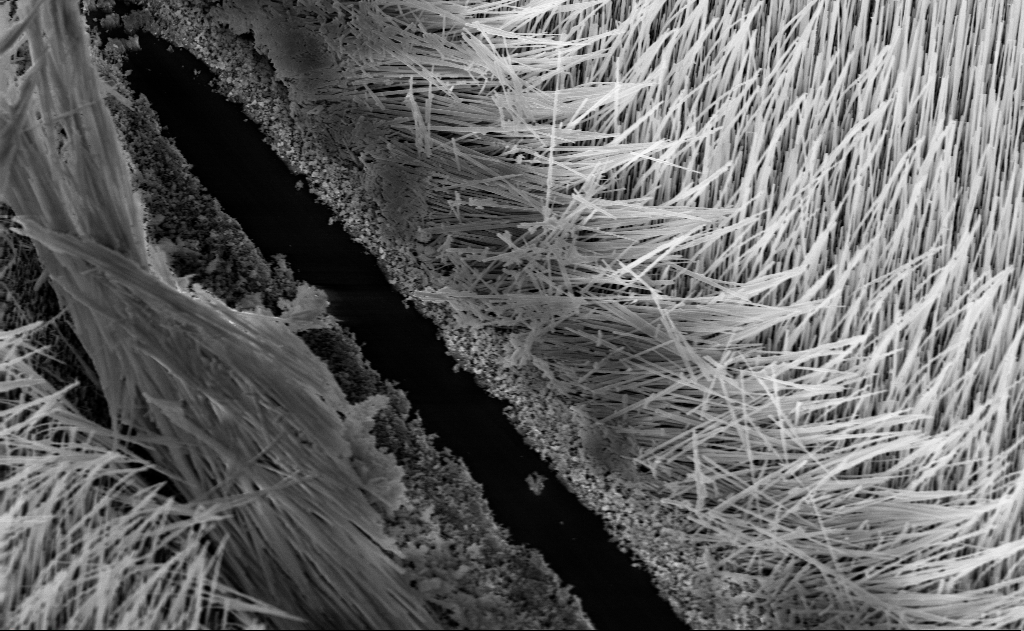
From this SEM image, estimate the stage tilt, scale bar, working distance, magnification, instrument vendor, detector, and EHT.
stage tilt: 30°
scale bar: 2000 nm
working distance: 9 mm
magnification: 8.56 K X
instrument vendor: Zeiss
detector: InLens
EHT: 10 kV